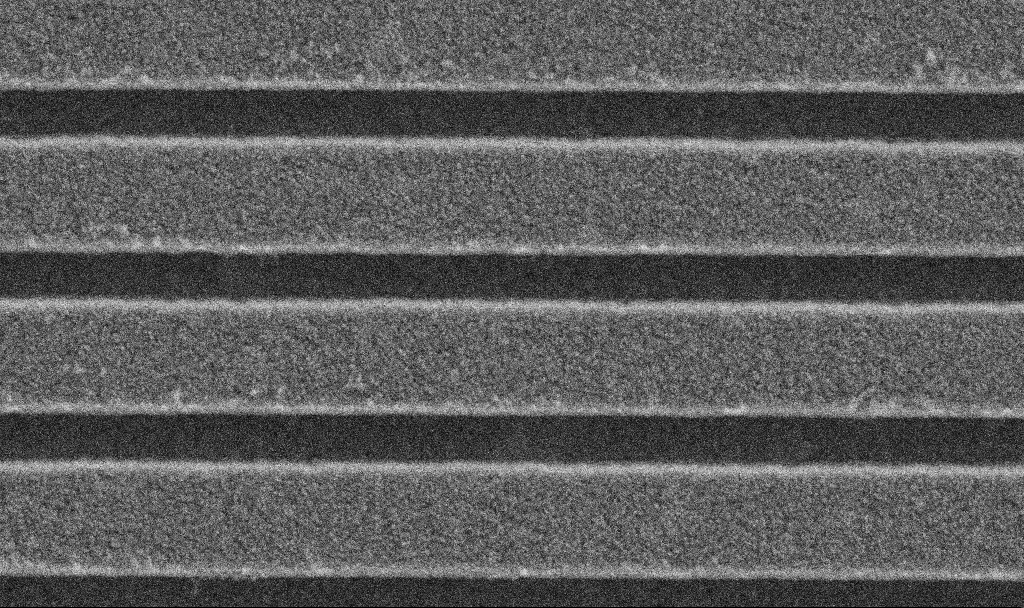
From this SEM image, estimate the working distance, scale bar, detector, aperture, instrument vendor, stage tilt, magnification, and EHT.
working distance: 2.9 mm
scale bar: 200 nm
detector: SE2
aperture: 30 µm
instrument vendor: Zeiss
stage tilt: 0°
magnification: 100.01 K X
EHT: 5 kV